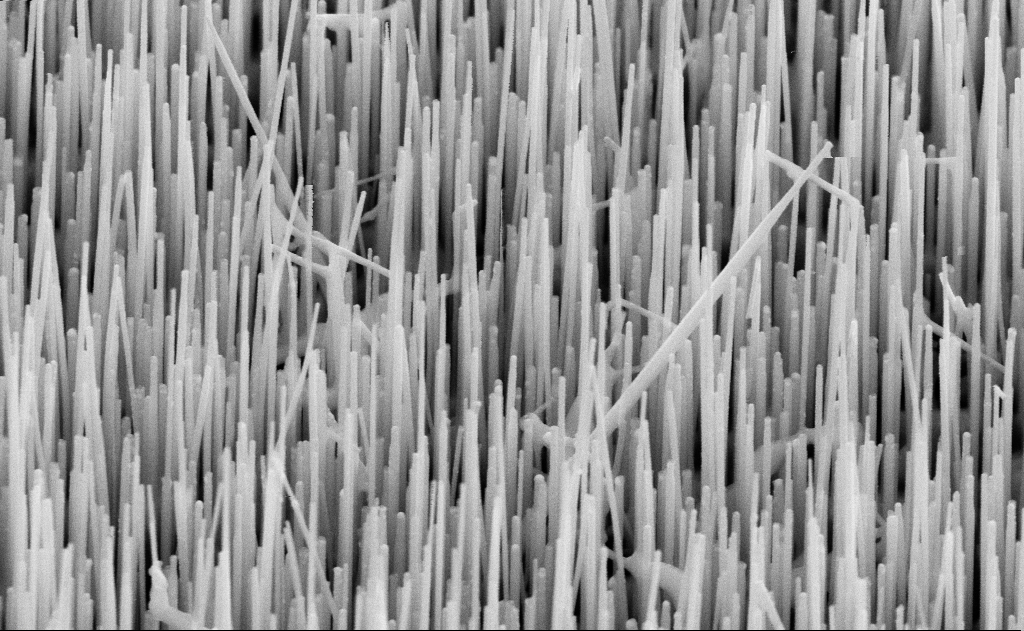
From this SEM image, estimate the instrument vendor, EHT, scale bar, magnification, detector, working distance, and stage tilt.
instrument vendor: Zeiss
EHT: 10 kV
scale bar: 1000 nm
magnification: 60 K X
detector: SE2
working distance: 11 mm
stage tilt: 45°